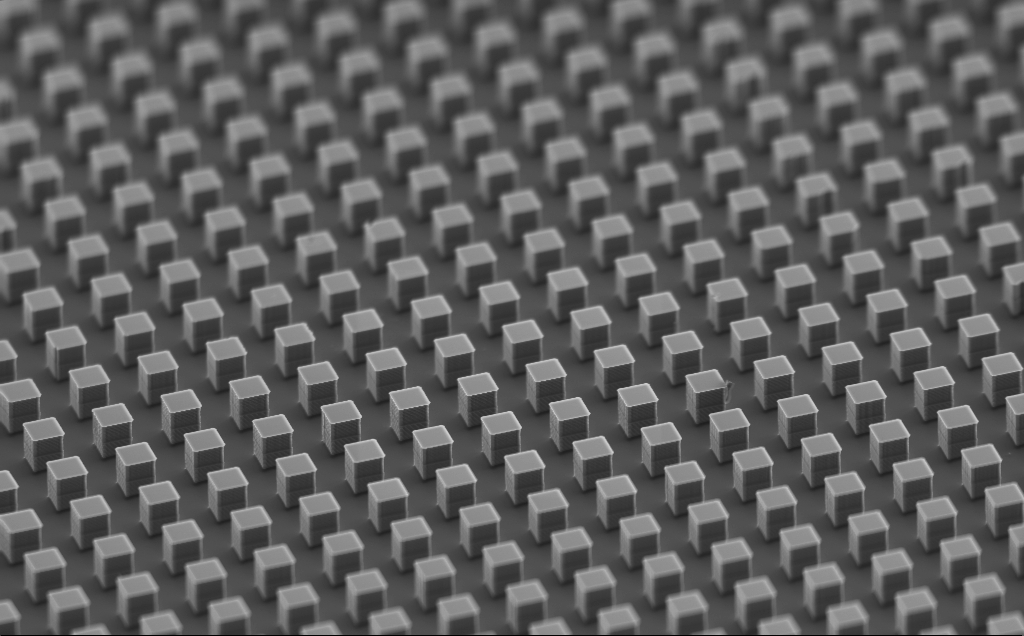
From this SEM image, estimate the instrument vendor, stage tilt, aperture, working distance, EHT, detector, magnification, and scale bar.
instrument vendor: Zeiss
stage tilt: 59.6°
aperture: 120 µm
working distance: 15 mm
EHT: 10 kV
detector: SE2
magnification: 1.32 K X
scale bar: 10000 nm